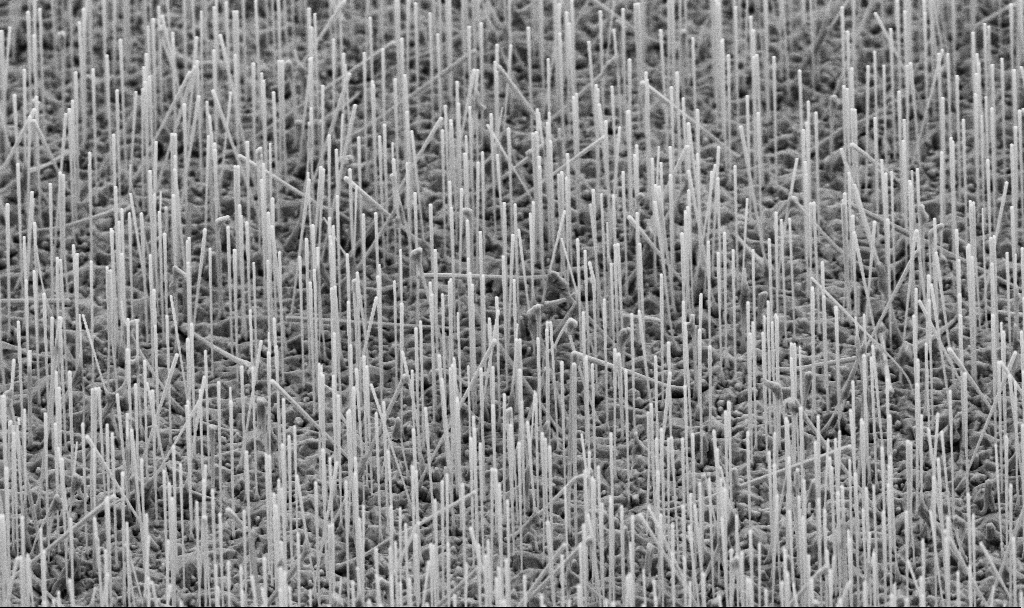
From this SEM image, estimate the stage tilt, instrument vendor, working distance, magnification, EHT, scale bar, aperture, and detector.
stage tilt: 45°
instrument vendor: Zeiss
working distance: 7.3 mm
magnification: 10 K X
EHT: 5 kV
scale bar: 2000 nm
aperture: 30 µm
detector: SE2